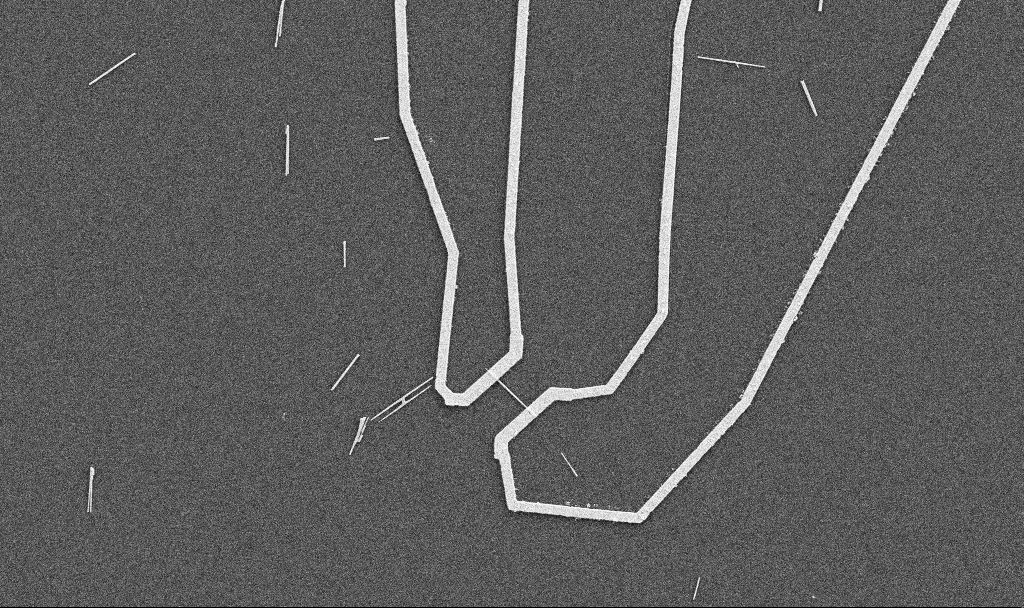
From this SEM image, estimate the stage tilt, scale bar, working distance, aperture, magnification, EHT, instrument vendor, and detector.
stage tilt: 0°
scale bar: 10000 nm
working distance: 10.7 mm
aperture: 30 µm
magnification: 5 K X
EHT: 5 kV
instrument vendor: Zeiss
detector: SE2